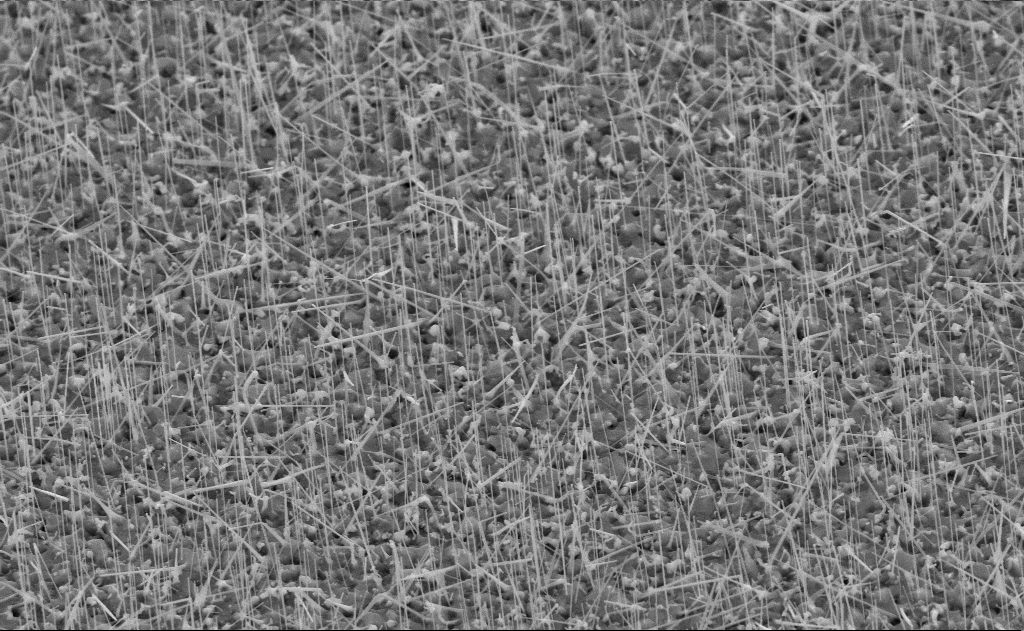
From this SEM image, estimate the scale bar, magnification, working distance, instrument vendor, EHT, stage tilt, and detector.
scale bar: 1000 nm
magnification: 20 K X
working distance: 11 mm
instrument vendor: Zeiss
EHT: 10 kV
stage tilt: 45°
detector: SE2